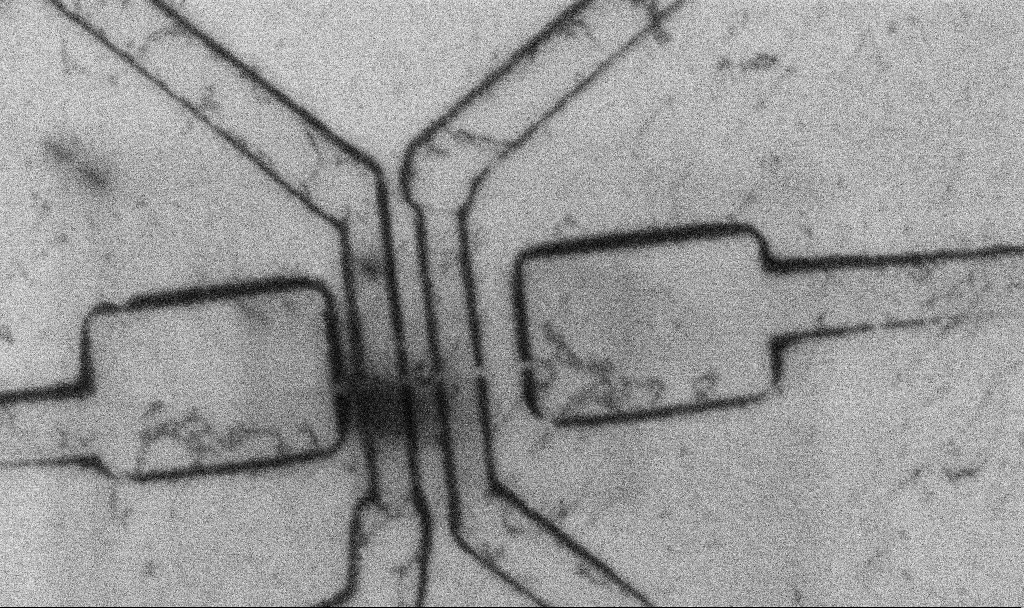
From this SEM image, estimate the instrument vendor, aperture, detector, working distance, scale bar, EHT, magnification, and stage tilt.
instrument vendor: Zeiss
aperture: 30 µm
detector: InLens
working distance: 9 mm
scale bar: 1000 nm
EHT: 5 kV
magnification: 27.57 K X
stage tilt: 0°